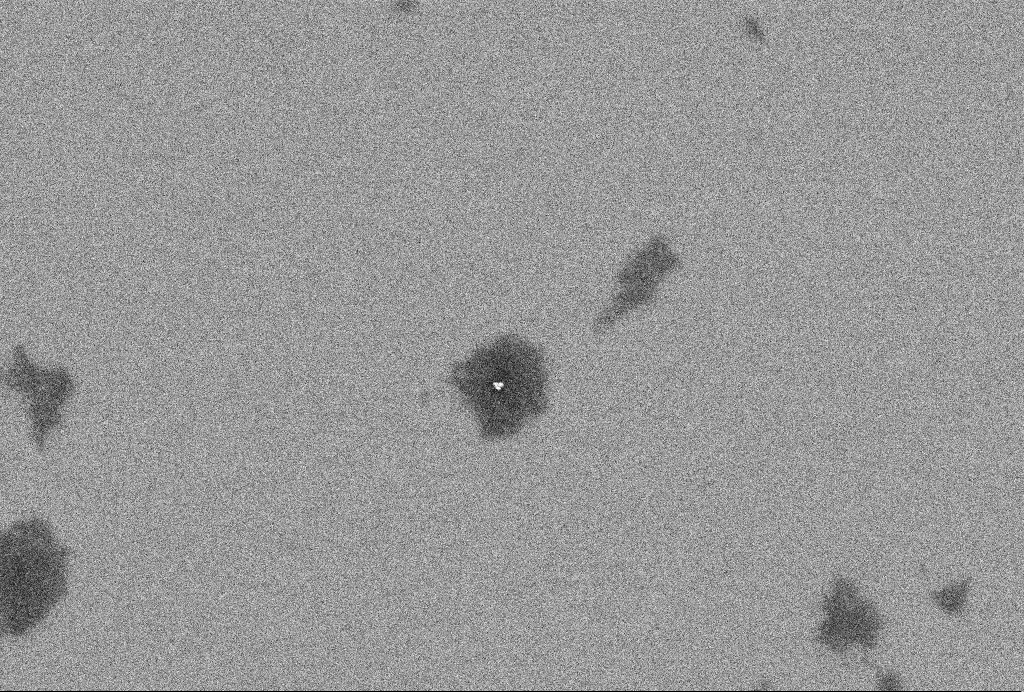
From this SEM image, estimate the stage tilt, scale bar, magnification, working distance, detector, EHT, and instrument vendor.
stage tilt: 0°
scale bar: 200 nm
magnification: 64.42 K X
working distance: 3.3 mm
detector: InLens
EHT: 2 kV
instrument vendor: Zeiss